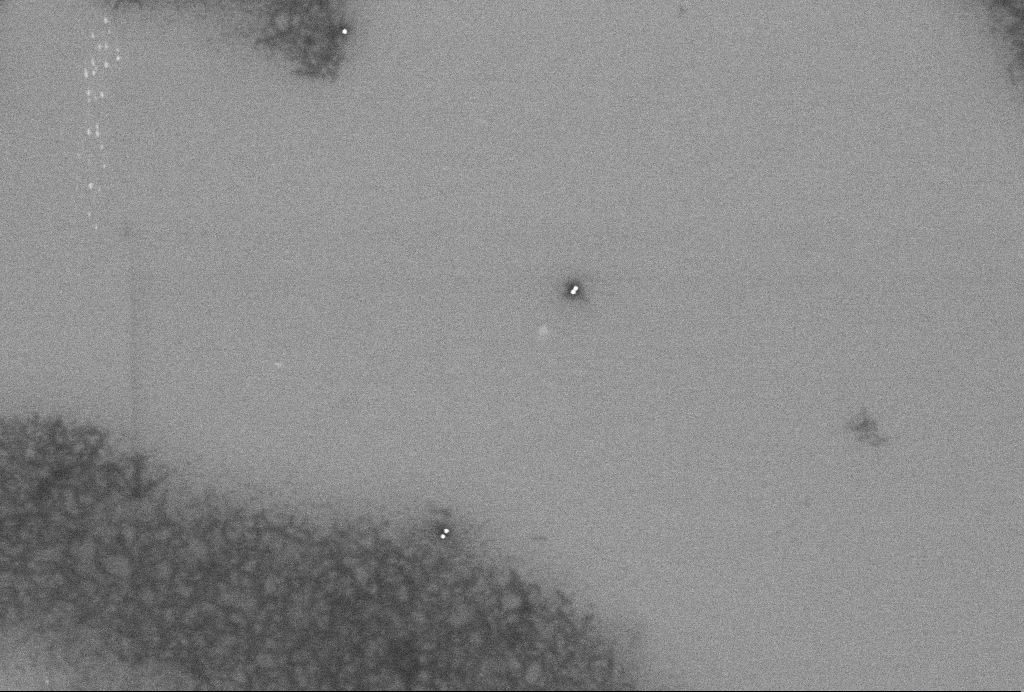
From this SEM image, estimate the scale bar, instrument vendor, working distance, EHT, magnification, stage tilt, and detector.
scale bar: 1000 nm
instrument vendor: Zeiss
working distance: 3.3 mm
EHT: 2 kV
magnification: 55.95 K X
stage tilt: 0°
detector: InLens